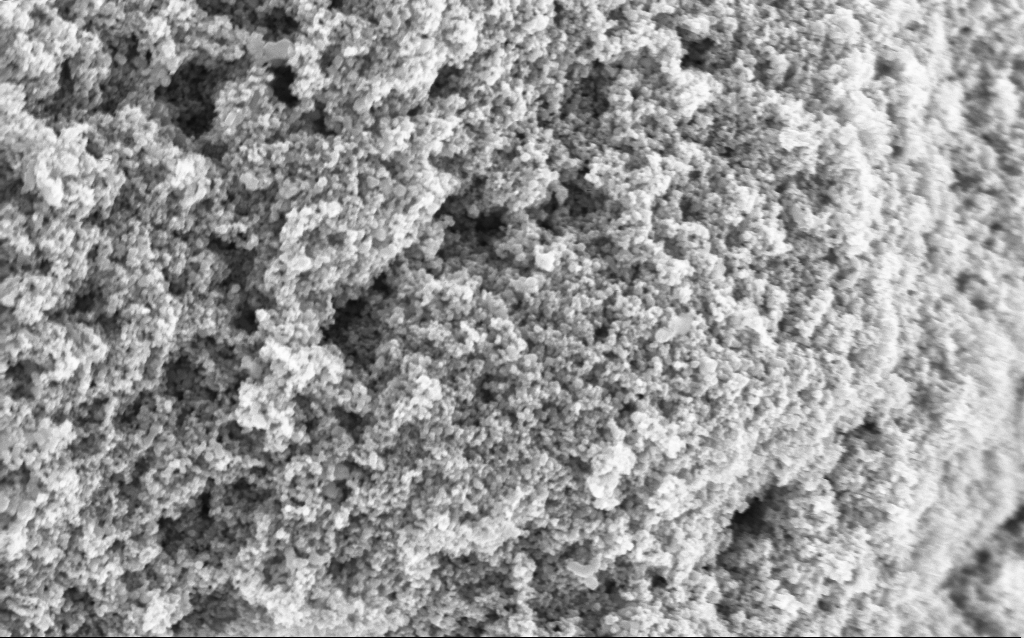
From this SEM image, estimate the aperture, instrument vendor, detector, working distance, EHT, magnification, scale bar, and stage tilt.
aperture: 30 µm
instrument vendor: Zeiss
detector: InLens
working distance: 4.5 mm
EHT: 5 kV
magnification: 68.64 K X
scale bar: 1000 nm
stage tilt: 0°